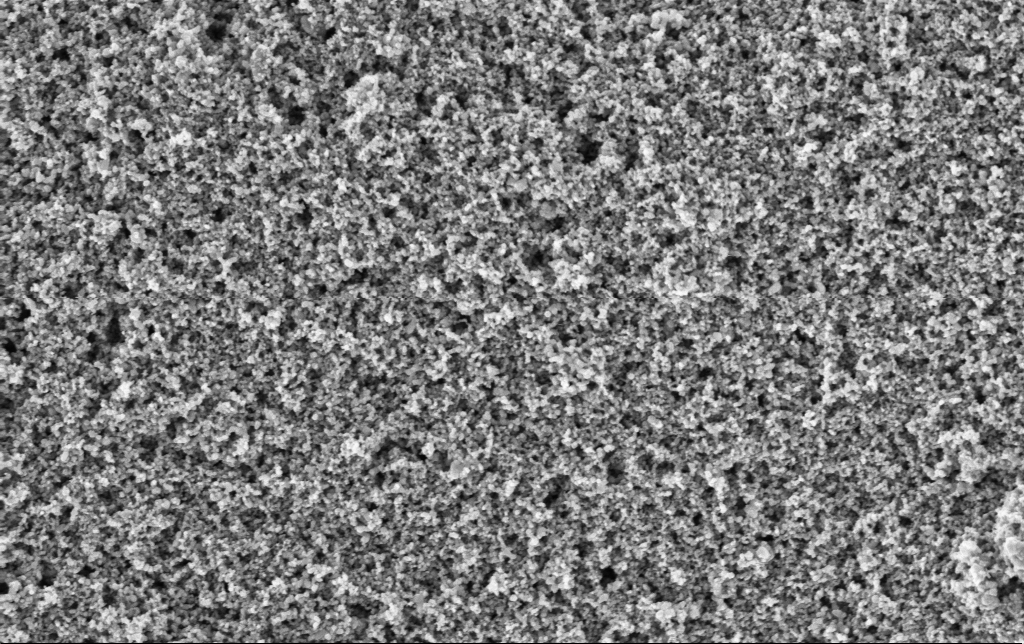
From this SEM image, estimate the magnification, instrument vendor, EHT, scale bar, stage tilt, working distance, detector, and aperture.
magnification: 50 K X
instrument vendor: Zeiss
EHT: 3 kV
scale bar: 1000 nm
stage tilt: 0°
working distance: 2.8 mm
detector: InLens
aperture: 30 µm